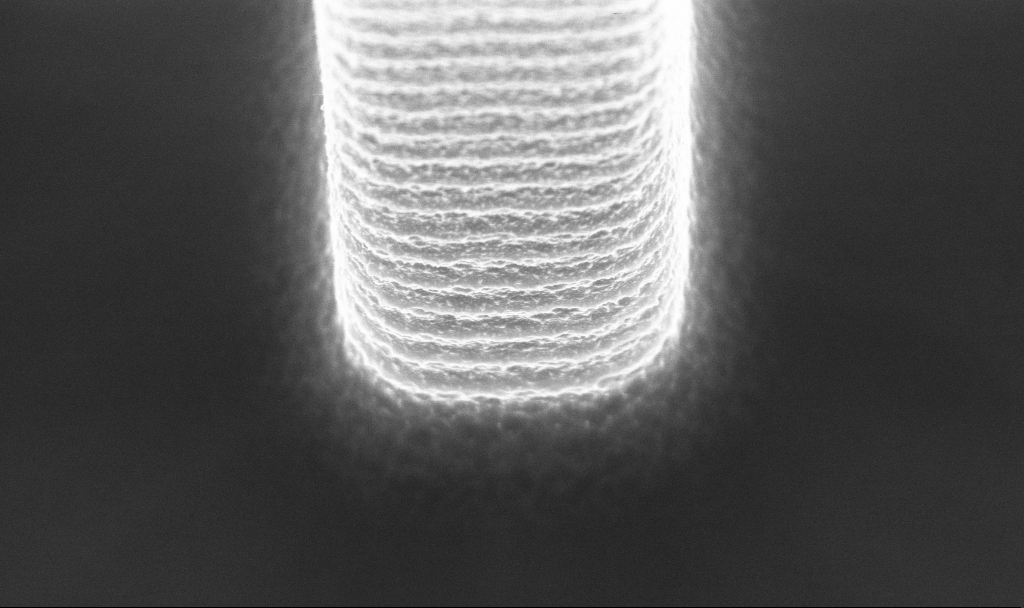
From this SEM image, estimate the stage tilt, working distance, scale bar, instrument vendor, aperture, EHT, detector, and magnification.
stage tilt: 20°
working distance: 4.2 mm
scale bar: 1000 nm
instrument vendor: Zeiss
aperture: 30 µm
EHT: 5 kV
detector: InLens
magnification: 62.4 K X